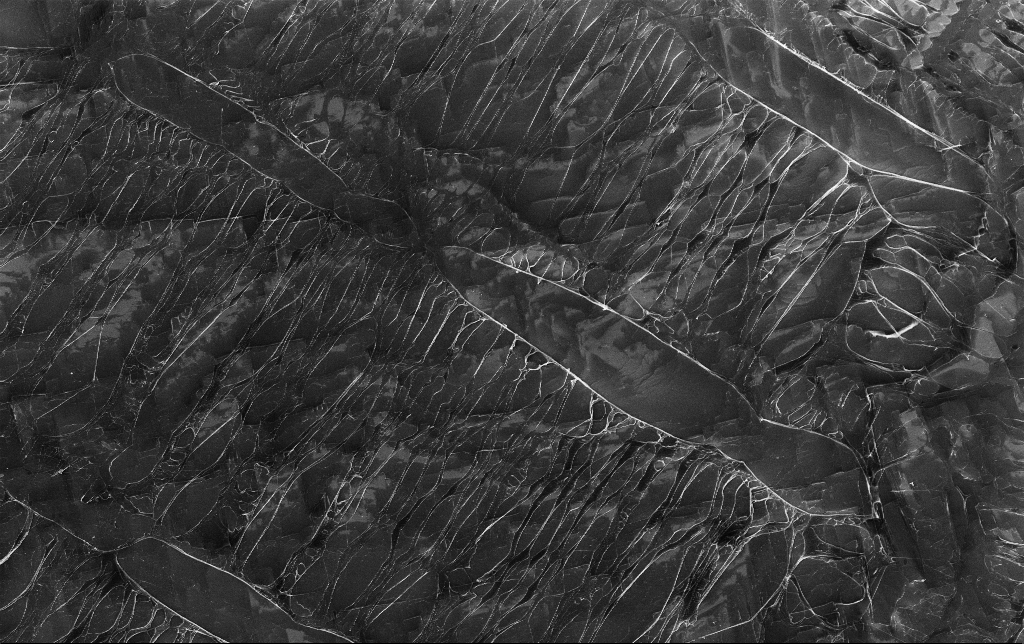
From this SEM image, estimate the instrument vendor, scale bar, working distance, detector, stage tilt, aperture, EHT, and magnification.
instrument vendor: Zeiss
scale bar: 20000 nm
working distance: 3.8 mm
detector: InLens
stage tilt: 0°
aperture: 30 µm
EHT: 5 kV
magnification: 1.17 K X